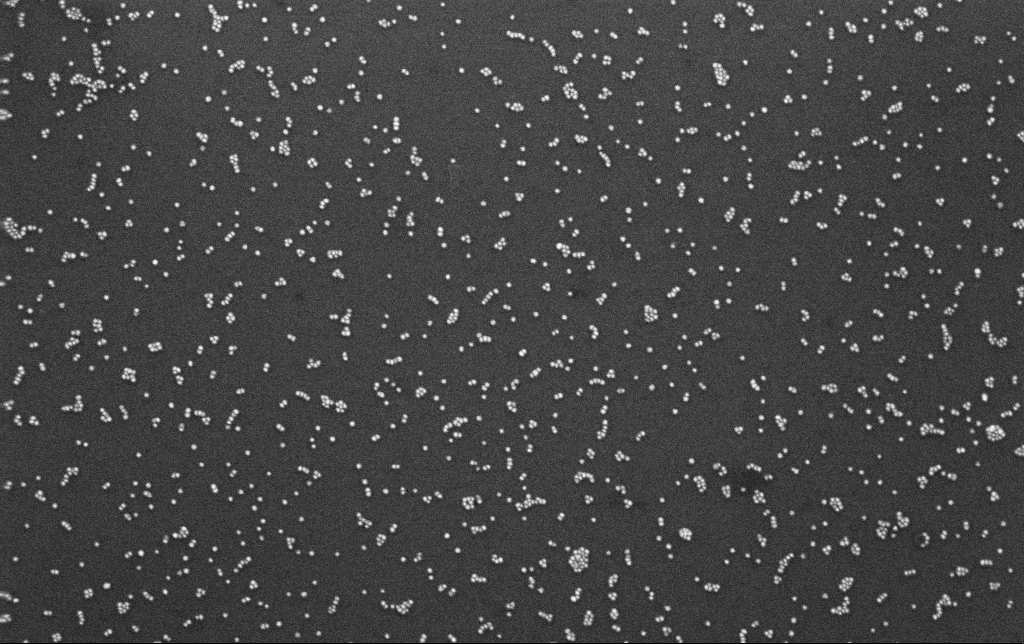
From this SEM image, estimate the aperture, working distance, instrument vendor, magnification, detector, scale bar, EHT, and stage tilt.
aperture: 30 µm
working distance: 3 mm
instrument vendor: Zeiss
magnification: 100 K X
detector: InLens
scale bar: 200 nm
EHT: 10 kV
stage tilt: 0°